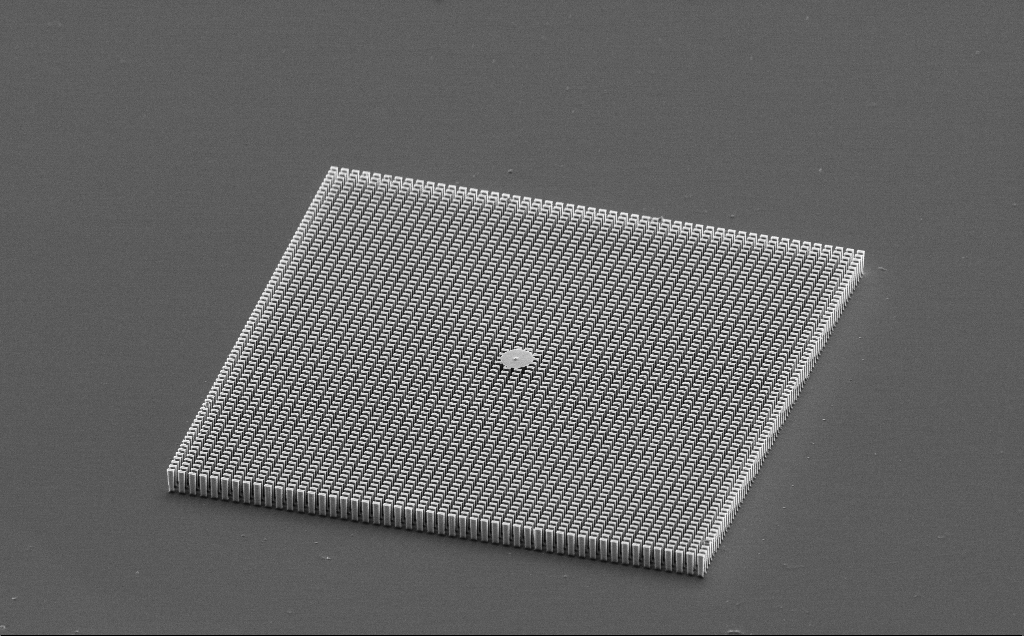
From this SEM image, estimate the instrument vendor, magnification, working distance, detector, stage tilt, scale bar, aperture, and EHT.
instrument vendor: Zeiss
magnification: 0.691 K X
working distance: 15 mm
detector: SE2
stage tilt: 60.2°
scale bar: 100000 nm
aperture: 30 µm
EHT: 10 kV